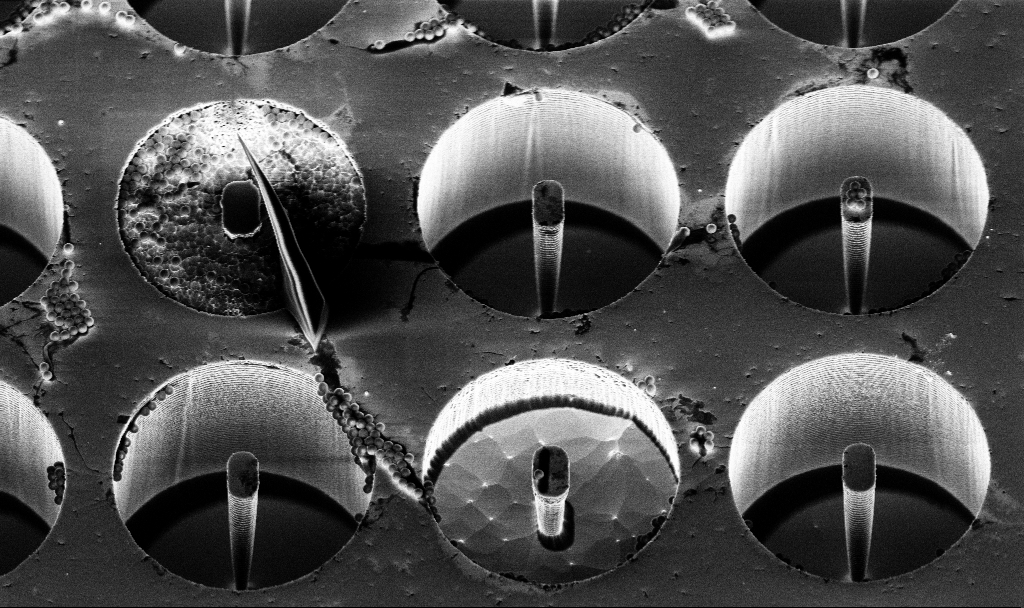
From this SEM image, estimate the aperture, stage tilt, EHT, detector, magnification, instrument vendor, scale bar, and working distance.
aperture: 30 µm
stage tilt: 30°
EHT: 5 kV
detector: InLens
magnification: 4.94 K X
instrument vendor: Zeiss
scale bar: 10000 nm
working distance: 7.1 mm